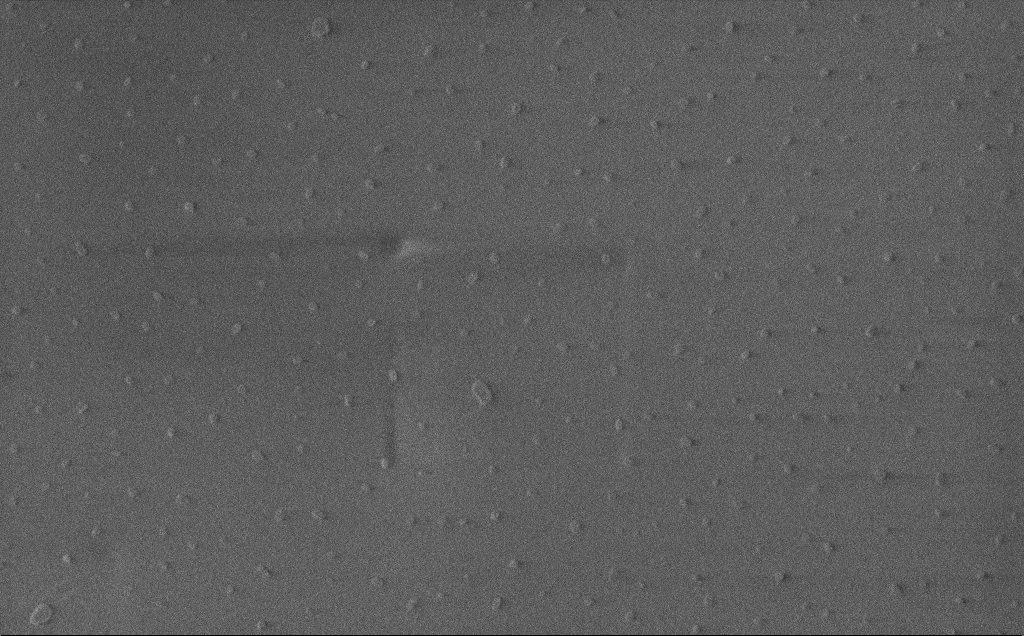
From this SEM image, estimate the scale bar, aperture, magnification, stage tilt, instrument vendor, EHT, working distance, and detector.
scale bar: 1000 nm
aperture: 30 µm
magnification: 45.29 K X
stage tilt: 0°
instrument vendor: Zeiss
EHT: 1 kV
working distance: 3 mm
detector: InLens